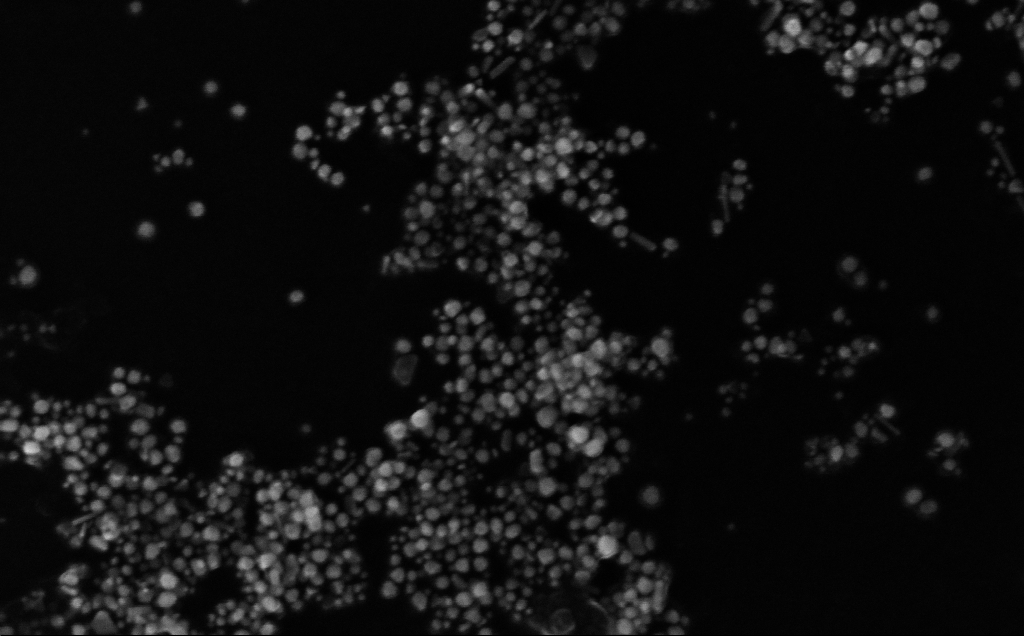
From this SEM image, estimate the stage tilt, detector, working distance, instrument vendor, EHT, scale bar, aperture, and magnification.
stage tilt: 0°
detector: InLens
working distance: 4 mm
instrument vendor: Zeiss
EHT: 10 kV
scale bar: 200 nm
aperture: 30 µm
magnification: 242.98 K X